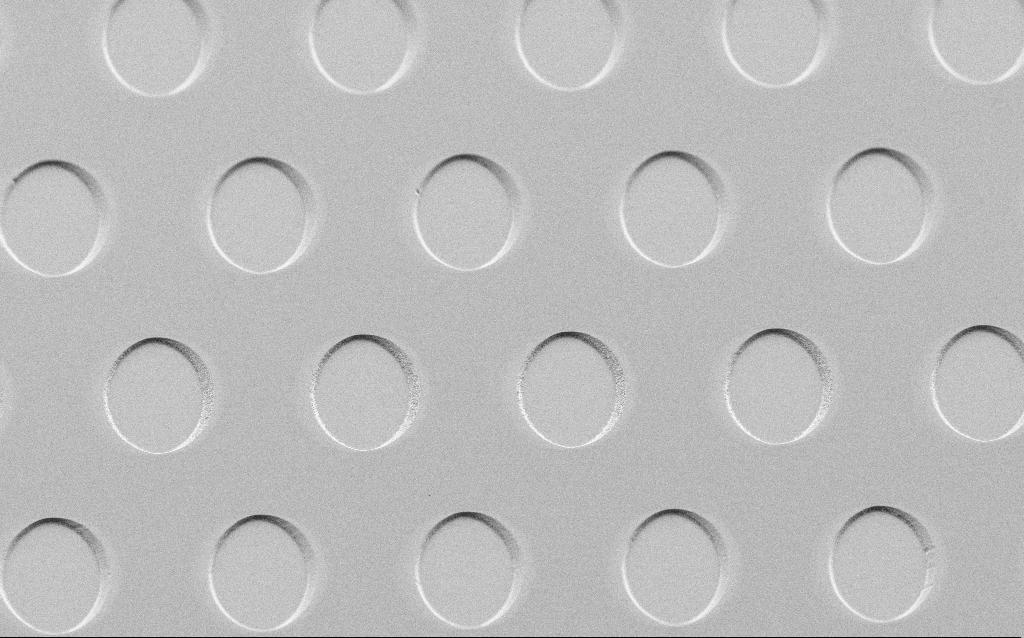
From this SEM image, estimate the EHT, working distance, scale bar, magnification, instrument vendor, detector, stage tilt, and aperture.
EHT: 3 kV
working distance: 6 mm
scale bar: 20000 nm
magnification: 1.1 K X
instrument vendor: Zeiss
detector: SE2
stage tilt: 45°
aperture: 30 µm